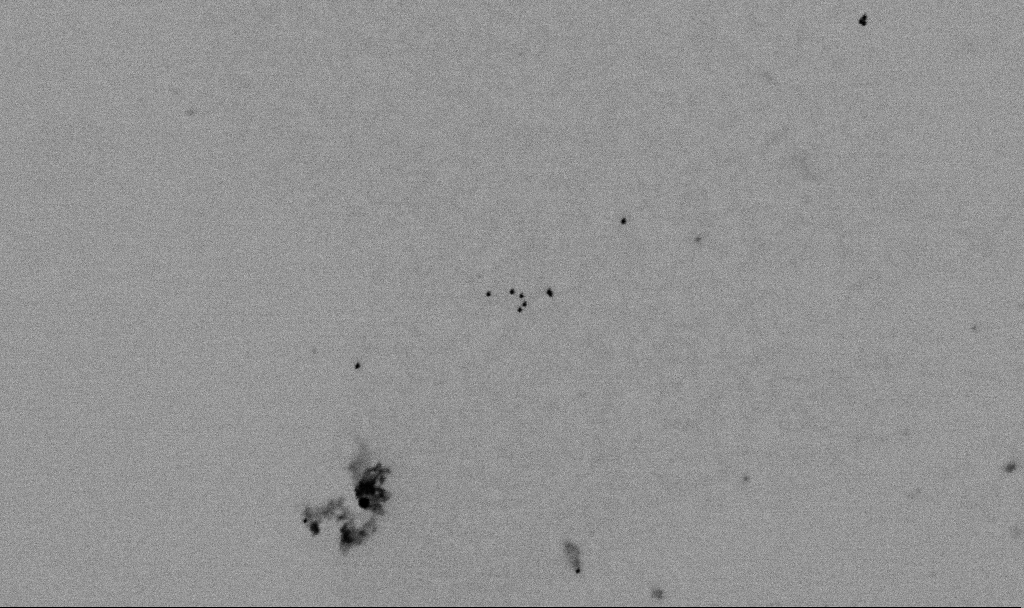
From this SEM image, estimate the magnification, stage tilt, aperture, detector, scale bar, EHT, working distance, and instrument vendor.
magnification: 60 K X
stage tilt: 0°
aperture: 30 µm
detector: SE2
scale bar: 1000 nm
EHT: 2 kV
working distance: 5.5 mm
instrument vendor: Zeiss